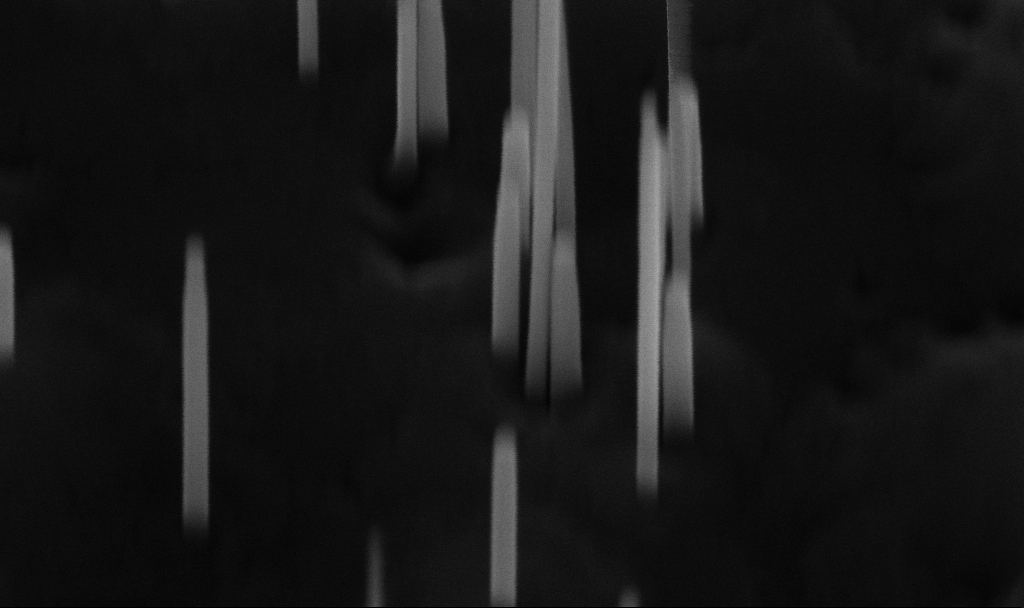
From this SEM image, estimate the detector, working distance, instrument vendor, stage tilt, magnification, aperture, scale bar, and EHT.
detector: InLens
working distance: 5.6 mm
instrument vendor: Zeiss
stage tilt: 45°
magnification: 280 K X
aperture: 30 µm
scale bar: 200 nm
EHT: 10 kV